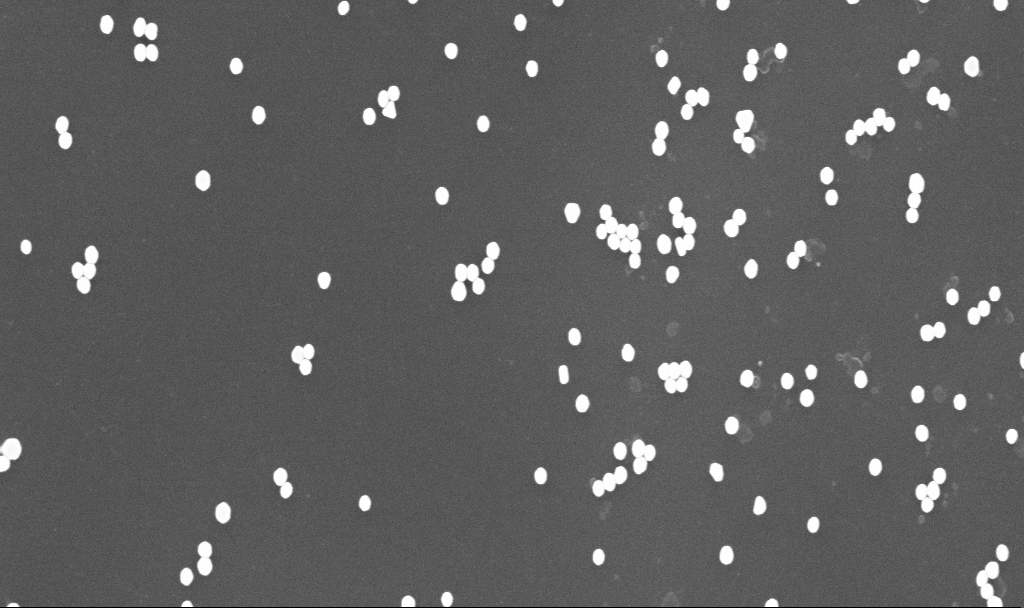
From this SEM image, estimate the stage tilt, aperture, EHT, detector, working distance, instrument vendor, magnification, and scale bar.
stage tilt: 0°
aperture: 30 µm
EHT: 10 kV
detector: InLens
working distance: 3.4 mm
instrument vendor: Zeiss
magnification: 70 K X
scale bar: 1000 nm